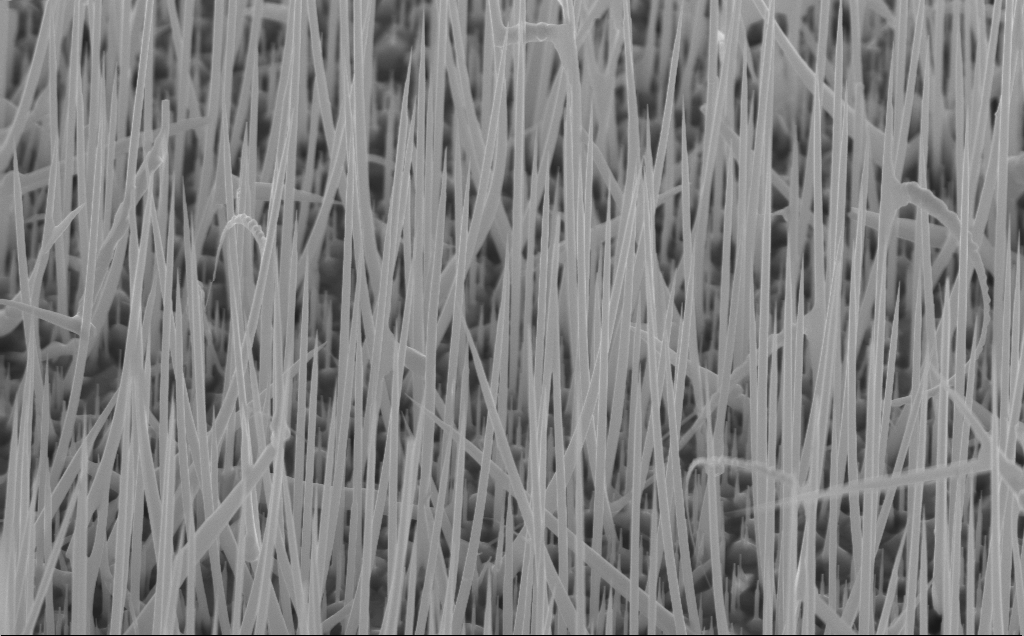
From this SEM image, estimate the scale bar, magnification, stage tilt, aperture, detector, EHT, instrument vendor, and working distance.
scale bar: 1000 nm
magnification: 27.71 K X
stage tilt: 45°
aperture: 30 µm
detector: InLens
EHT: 10 kV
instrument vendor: Zeiss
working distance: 4 mm